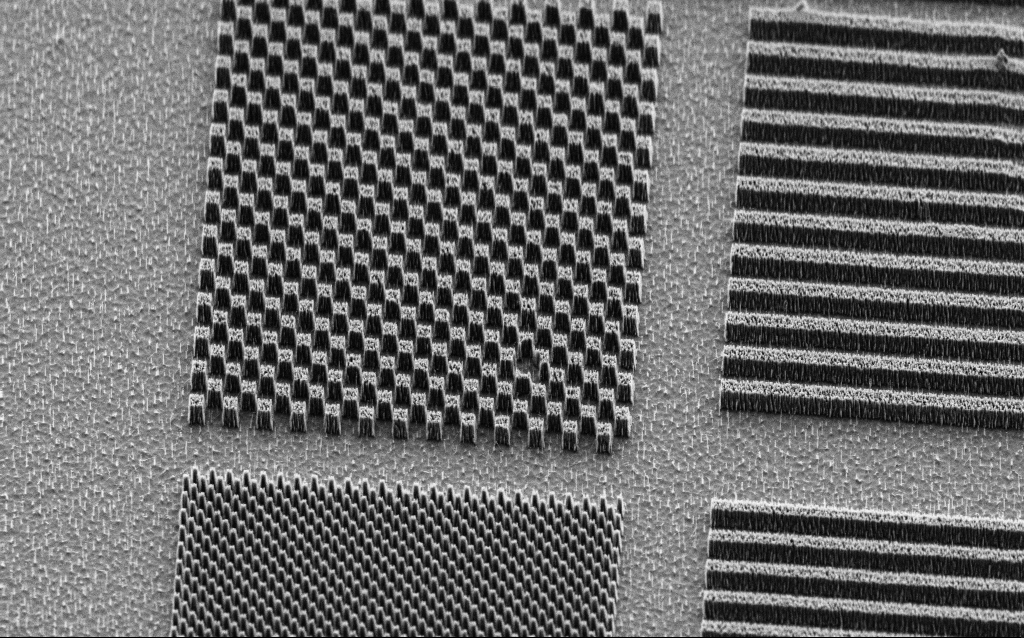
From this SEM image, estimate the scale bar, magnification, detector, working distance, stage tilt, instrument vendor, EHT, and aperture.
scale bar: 10000 nm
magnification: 6.28 K X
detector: SE2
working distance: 8 mm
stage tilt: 45°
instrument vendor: Zeiss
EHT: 3 kV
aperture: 30 µm